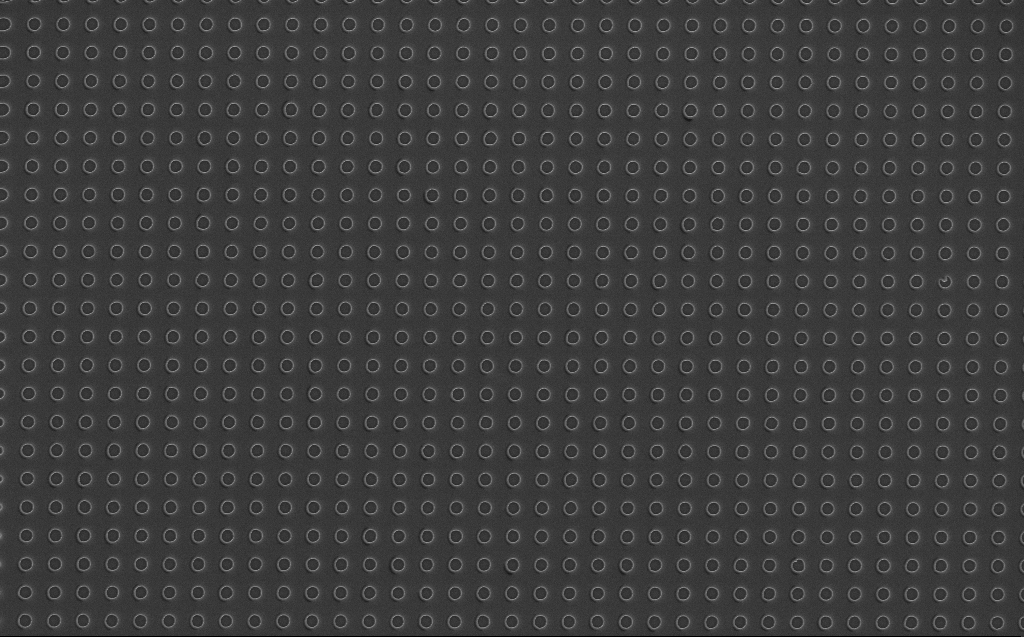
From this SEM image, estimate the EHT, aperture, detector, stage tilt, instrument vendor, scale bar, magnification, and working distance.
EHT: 5 kV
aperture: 30 µm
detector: InLens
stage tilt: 0°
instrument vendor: Zeiss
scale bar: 2000 nm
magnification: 10 K X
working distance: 7 mm